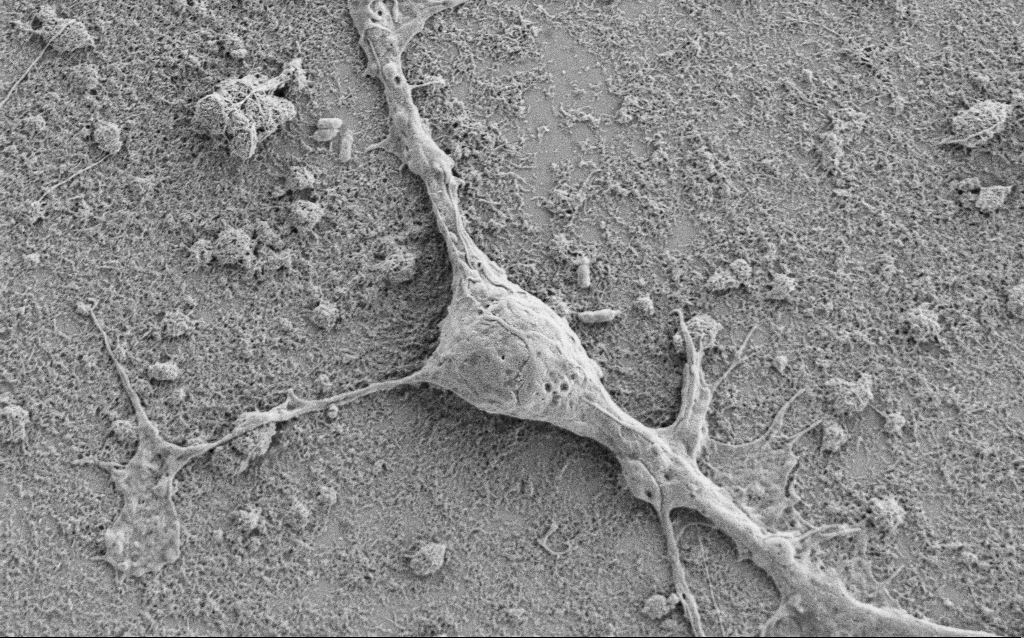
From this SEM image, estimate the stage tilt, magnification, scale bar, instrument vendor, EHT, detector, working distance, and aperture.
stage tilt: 0°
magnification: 7.5 K X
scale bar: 2000 nm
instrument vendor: Zeiss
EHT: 1.5 kV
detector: SE2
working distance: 6.8 mm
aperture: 30 µm